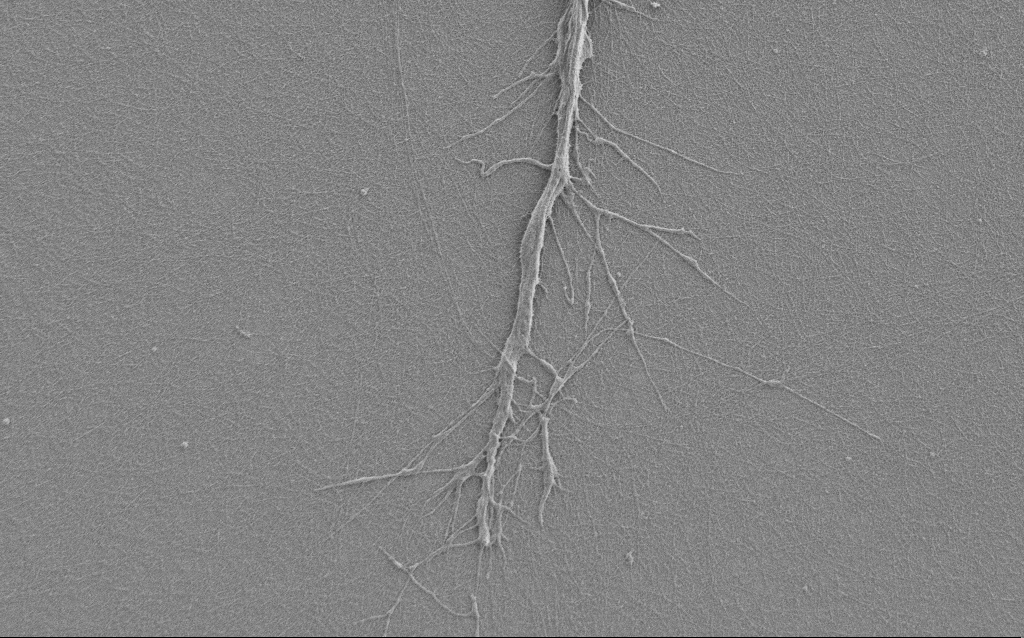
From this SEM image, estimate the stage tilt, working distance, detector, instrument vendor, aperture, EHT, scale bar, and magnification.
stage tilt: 0°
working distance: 6 mm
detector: SE2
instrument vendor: Zeiss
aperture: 30 µm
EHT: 1 kV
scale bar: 10000 nm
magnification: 6 K X